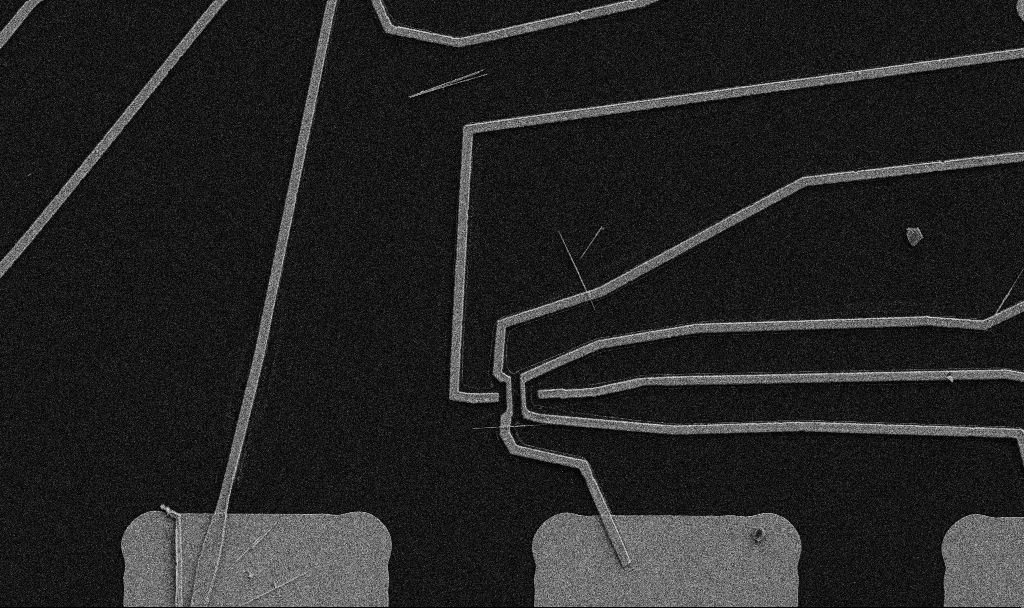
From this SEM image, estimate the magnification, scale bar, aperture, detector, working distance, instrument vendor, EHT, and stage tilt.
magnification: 5 K X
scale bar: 10000 nm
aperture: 30 µm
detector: SE2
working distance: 10.7 mm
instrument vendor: Zeiss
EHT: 5 kV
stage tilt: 0°